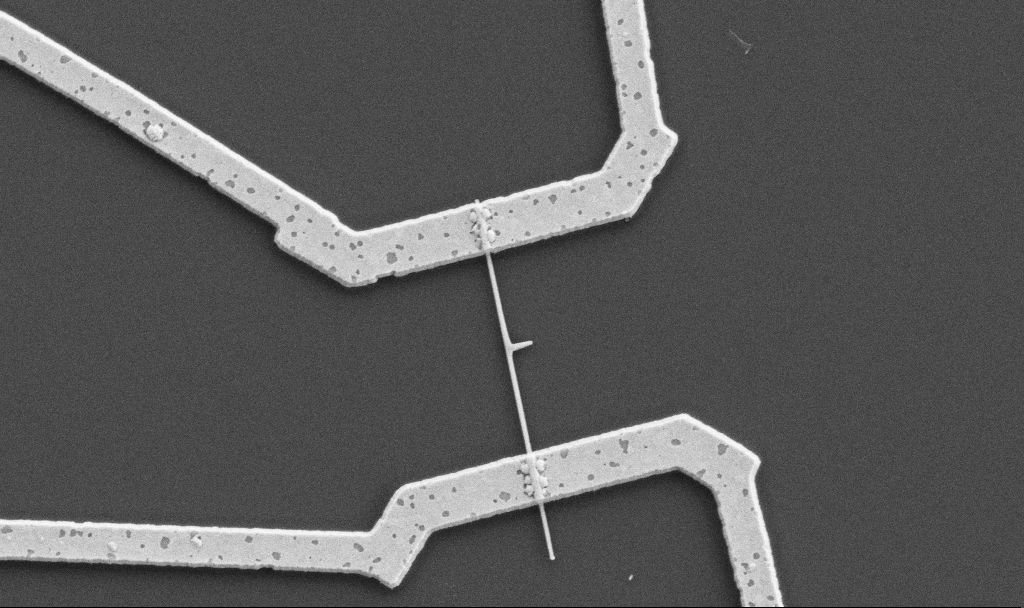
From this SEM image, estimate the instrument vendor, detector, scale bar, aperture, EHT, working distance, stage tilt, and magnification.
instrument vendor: Zeiss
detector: SE2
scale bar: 1000 nm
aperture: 30 µm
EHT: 5 kV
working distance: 10.7 mm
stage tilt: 0°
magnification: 20 K X